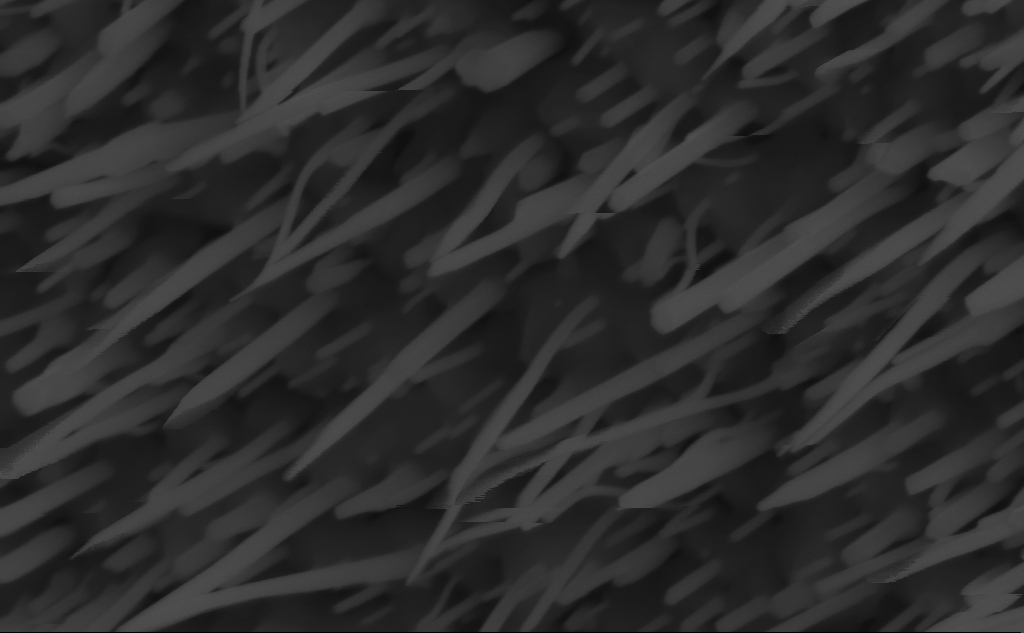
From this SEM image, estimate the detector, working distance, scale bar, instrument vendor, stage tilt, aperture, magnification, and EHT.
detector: InLens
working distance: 6 mm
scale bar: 200 nm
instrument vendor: Zeiss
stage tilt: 45°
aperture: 30 µm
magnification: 159.1 K X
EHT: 10 kV